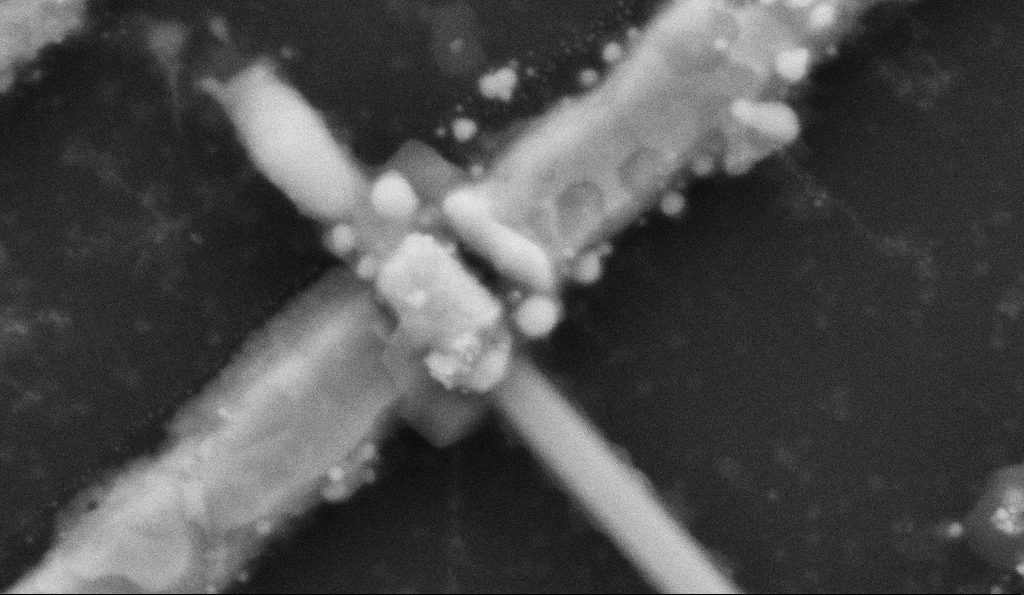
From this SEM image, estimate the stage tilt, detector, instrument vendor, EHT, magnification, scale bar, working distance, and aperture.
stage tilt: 0°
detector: SE2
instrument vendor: Zeiss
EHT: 5 kV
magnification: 200 K X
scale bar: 200 nm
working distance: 8.5 mm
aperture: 30 µm